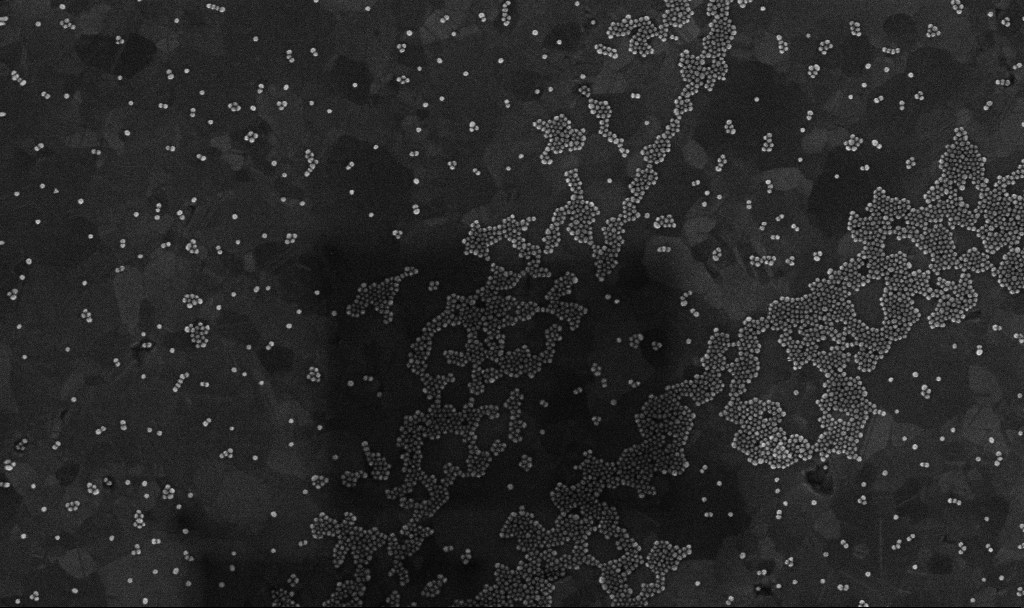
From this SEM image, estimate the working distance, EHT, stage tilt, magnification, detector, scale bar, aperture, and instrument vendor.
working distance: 3.4 mm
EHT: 10 kV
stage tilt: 0°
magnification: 100 K X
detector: InLens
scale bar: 200 nm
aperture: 30 µm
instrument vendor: Zeiss